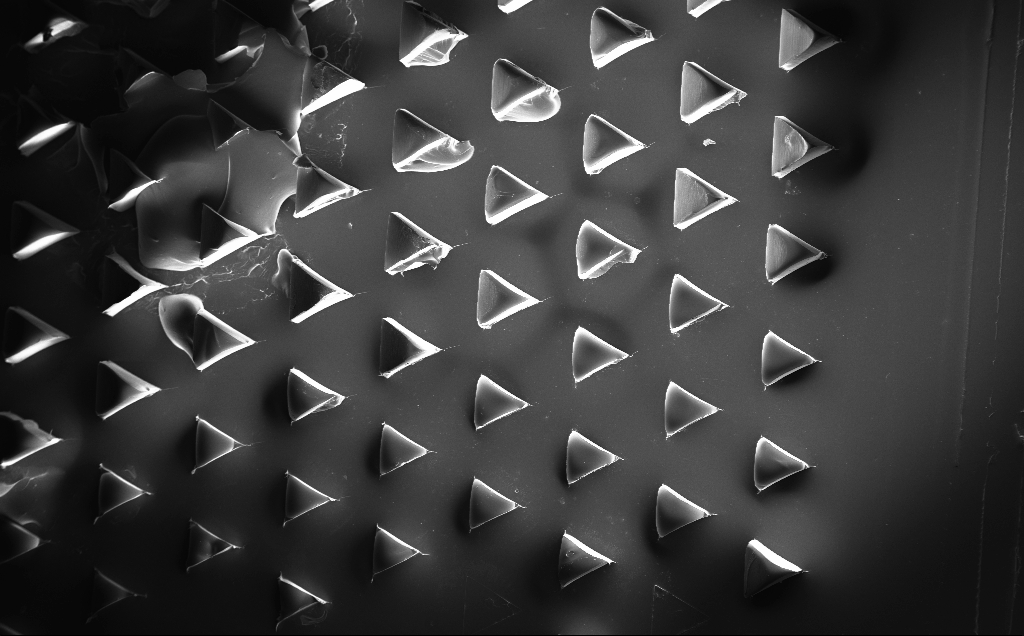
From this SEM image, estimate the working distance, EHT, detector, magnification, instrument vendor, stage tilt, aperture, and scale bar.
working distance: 10 mm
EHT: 10 kV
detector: InLens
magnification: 0.091 K X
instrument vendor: Zeiss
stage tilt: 0°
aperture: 30 µm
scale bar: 200000 nm